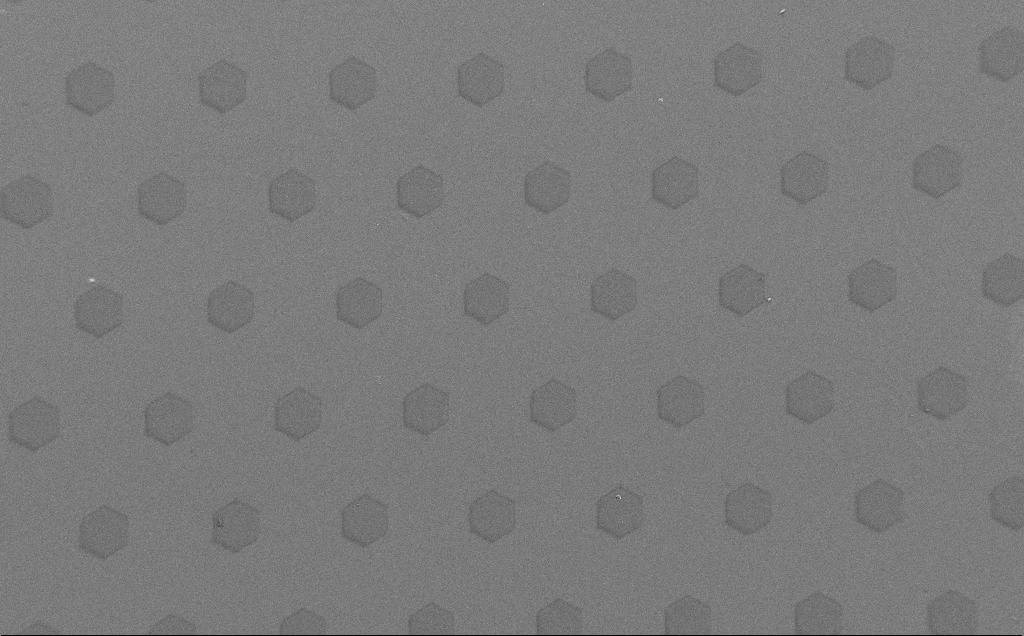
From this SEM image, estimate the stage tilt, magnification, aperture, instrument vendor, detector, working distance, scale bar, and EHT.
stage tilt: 0°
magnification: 0.308 K X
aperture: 30 µm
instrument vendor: Zeiss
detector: SE2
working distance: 7 mm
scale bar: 100000 nm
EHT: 1.5 kV